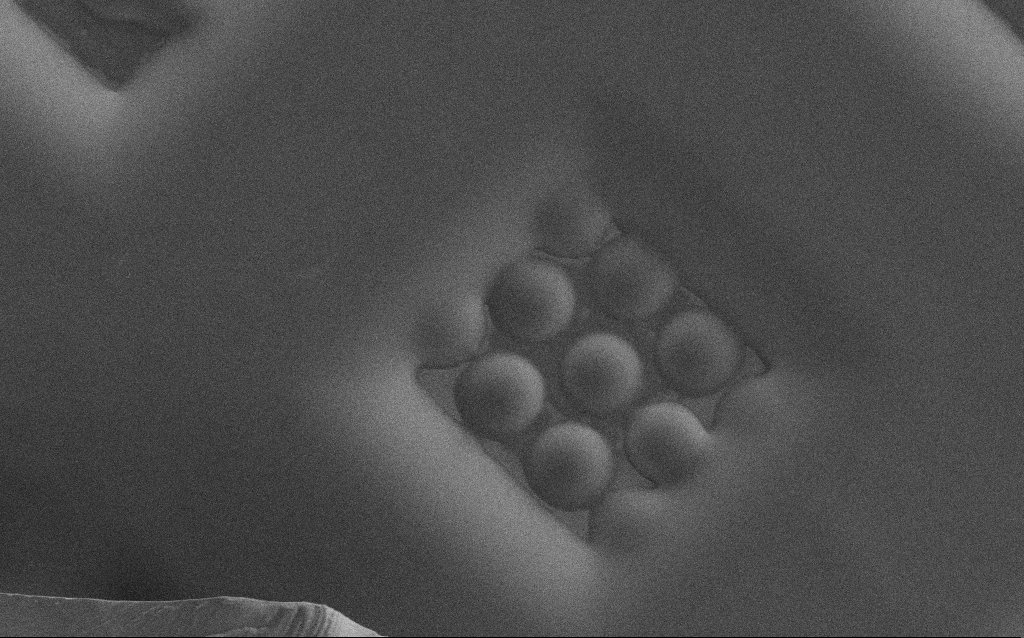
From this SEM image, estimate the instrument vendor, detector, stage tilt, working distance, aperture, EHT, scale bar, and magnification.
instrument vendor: Zeiss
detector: SE2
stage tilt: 0°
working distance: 7 mm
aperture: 30 µm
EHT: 1.5 kV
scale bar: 10000 nm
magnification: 1.71 K X